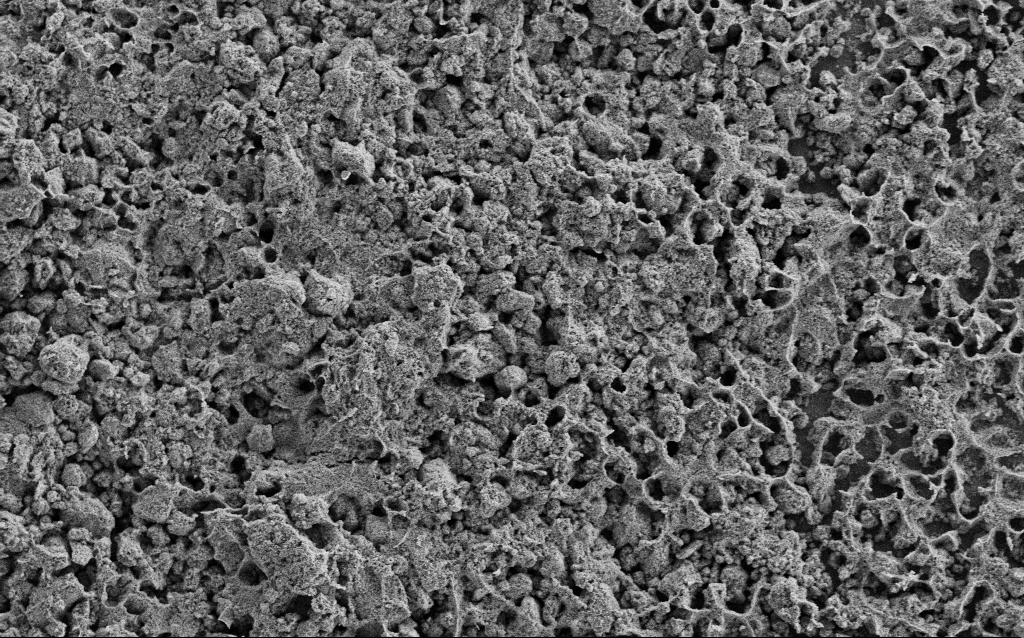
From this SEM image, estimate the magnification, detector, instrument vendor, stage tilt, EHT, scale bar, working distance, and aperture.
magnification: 1.23 K X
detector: SE2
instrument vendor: Zeiss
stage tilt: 0°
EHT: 5 kV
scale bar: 10000 nm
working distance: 4.4 mm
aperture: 30 µm